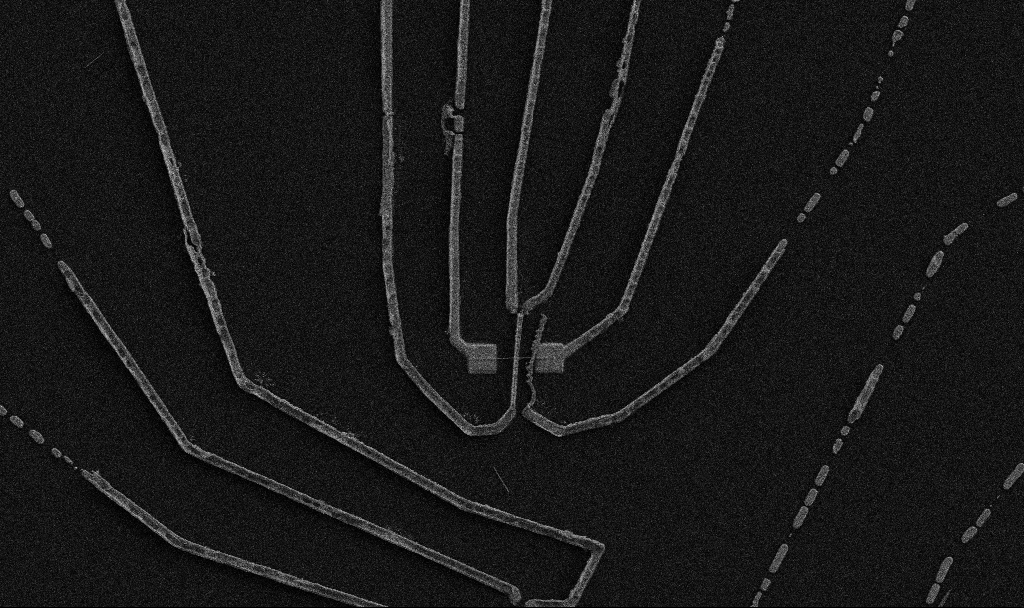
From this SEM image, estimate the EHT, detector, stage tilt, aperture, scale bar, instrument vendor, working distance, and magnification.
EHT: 5 kV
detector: SE2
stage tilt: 0°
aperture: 30 µm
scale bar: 10000 nm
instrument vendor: Zeiss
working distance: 10.7 mm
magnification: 5 K X